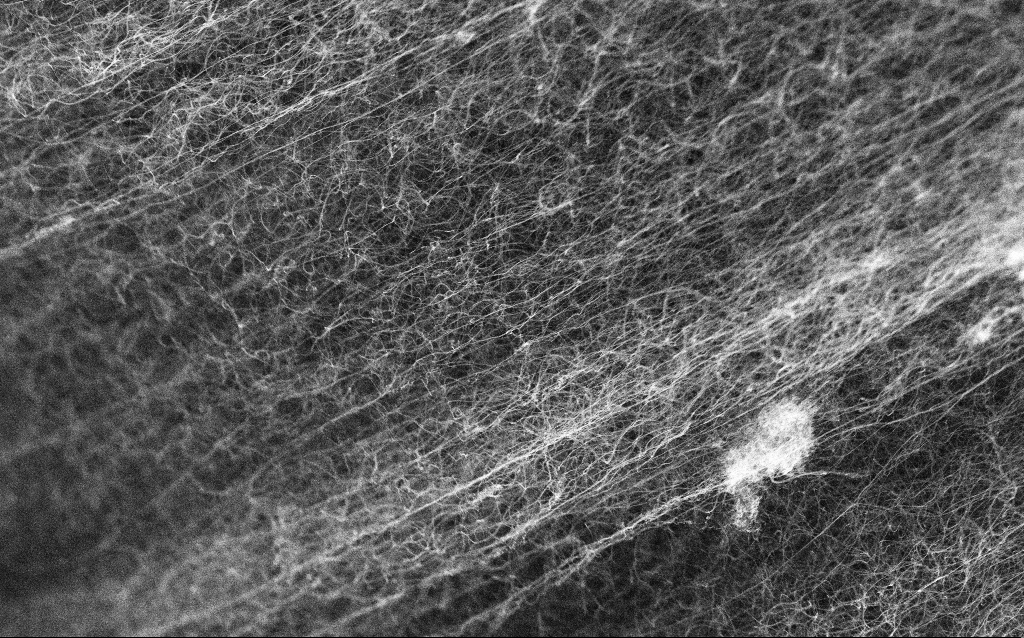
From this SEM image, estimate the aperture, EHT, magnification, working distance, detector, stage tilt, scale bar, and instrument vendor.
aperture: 30 µm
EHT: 5 kV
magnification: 20 K X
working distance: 2.3 mm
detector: InLens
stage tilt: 0°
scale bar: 1000 nm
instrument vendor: Zeiss